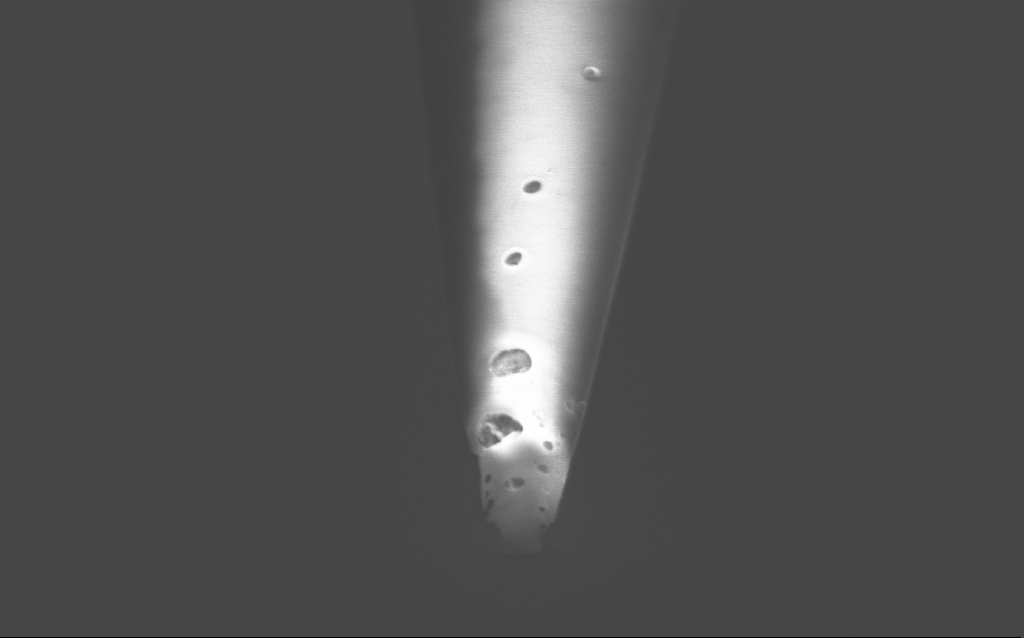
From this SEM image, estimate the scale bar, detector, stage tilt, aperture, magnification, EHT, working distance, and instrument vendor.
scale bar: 200 nm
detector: InLens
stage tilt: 45°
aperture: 30 µm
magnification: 75 K X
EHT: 2 kV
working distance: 6 mm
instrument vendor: Zeiss